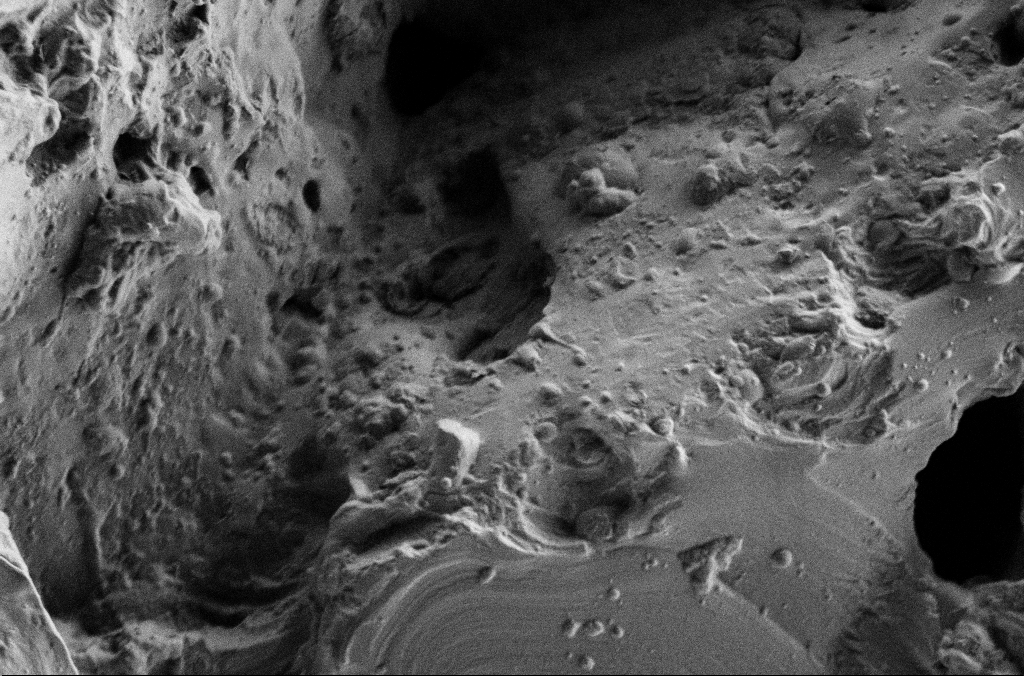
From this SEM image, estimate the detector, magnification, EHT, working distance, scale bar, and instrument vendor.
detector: SE2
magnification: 5 K X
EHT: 2 kV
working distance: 3 mm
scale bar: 10000 nm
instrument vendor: Zeiss